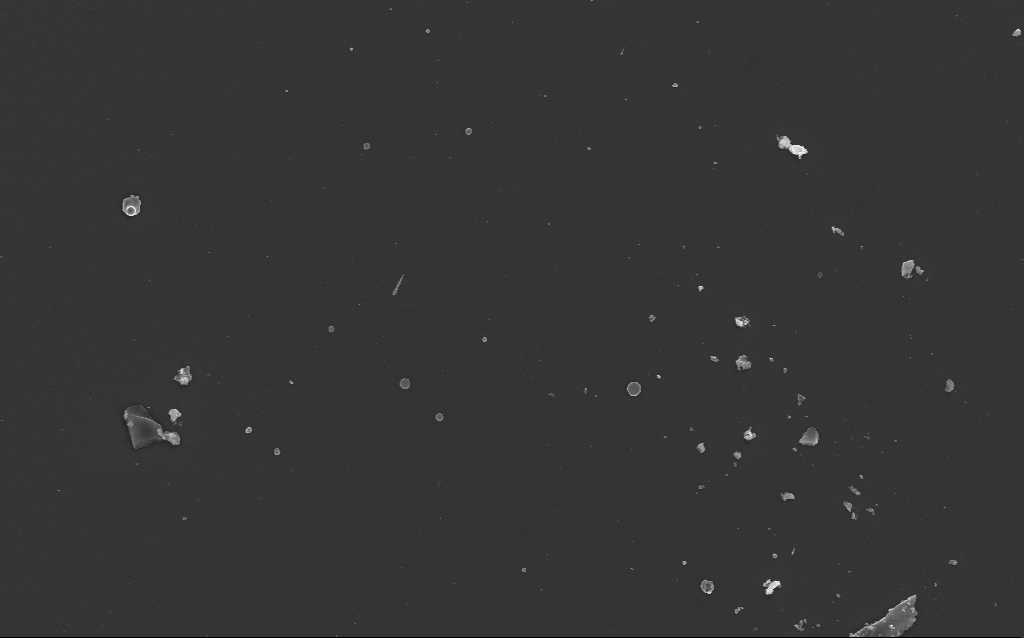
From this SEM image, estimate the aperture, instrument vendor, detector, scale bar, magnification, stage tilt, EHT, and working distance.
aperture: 30 µm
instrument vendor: Zeiss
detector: InLens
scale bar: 10000 nm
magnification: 4.74 K X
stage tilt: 0°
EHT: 10 kV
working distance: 3 mm